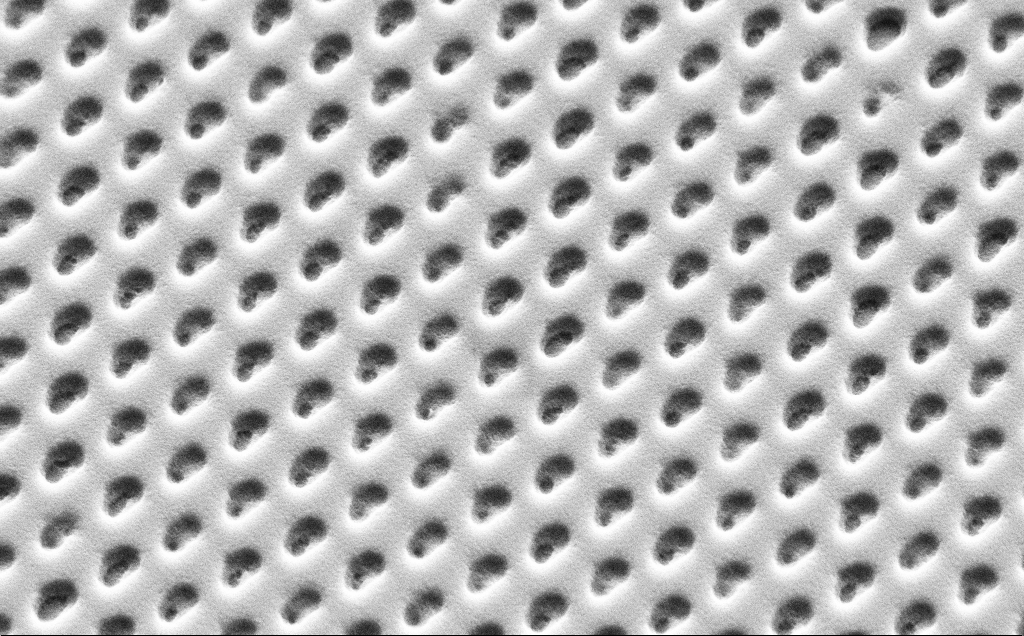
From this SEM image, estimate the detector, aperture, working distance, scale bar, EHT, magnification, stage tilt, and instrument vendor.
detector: SE2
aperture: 30 µm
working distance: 10 mm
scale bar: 1000 nm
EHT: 5 kV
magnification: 12.91 K X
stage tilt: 45°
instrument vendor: Zeiss